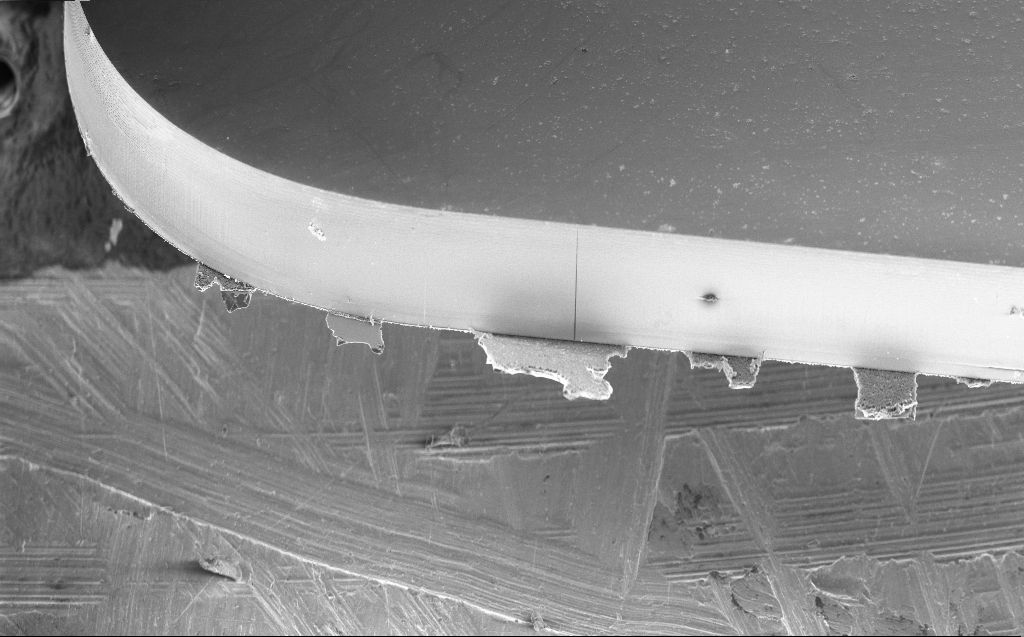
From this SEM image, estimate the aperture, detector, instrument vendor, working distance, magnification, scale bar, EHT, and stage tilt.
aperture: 30 µm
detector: InLens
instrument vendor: Zeiss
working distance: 5 mm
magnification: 0.471 K X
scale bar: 100000 nm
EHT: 5 kV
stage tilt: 45°